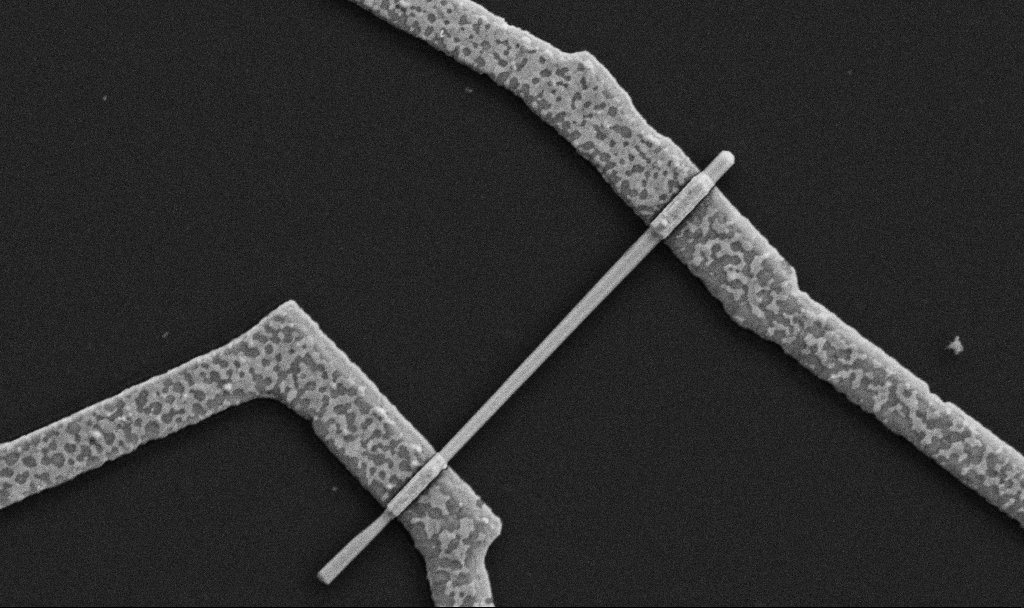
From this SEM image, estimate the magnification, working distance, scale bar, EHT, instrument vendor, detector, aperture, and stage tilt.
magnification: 30 K X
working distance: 8.7 mm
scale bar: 1000 nm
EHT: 5 kV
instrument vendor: Zeiss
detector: SE2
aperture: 30 µm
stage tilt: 0°